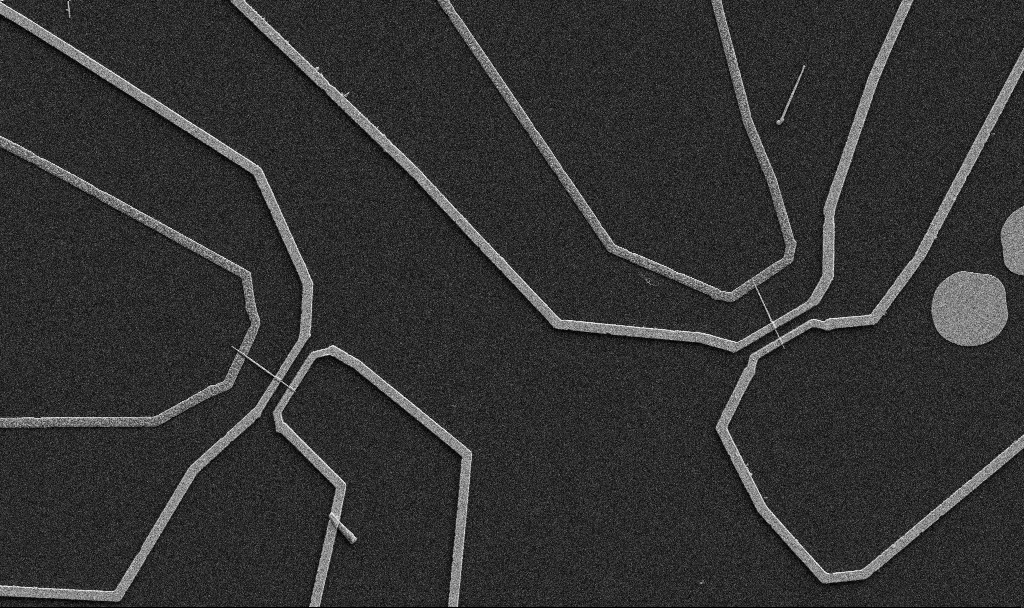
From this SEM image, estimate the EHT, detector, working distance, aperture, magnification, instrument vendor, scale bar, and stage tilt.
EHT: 5 kV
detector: SE2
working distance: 10.7 mm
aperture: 30 µm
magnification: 5 K X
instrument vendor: Zeiss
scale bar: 10000 nm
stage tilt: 0°